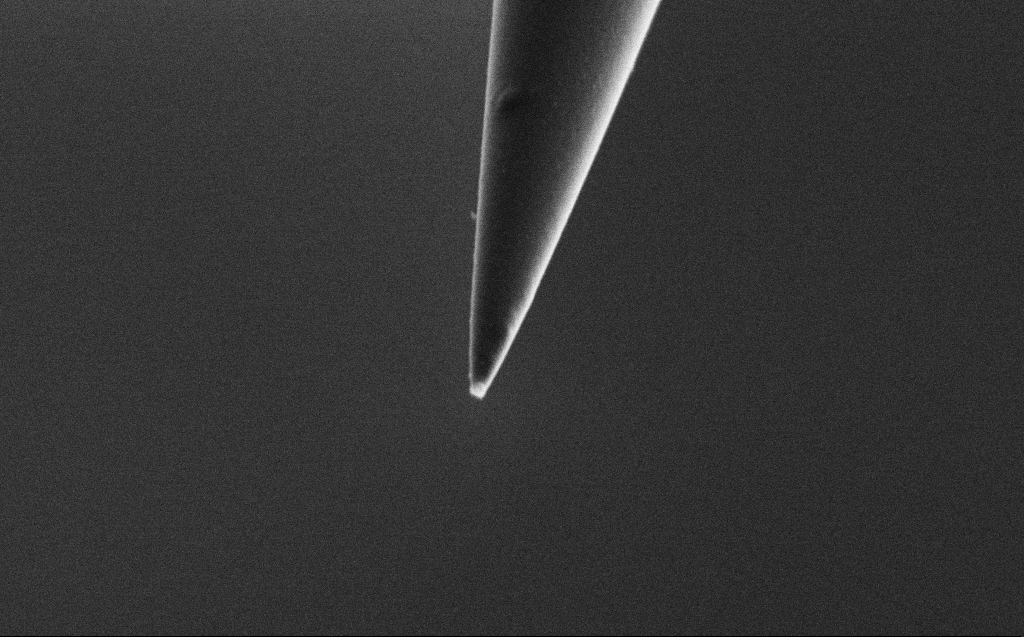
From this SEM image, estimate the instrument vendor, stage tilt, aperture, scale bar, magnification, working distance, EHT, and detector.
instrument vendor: Zeiss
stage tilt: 45°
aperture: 30 µm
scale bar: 1000 nm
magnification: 50 K X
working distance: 4 mm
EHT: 2 kV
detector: SE2